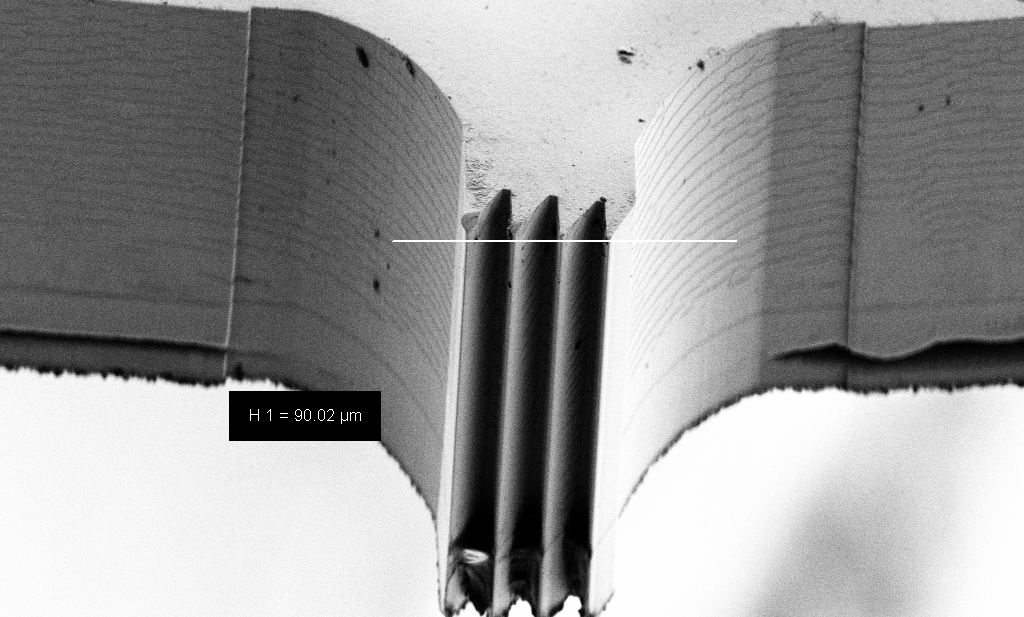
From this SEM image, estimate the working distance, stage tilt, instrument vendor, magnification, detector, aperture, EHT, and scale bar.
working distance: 8 mm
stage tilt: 45°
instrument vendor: Zeiss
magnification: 1.54 K X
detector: SE2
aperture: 30 µm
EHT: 3 kV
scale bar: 20000 nm